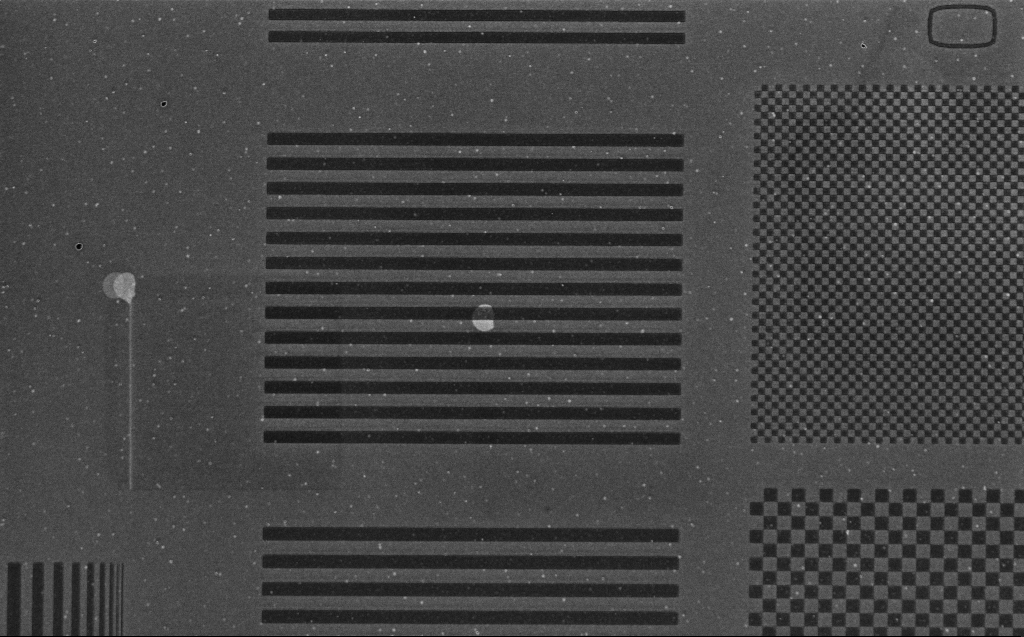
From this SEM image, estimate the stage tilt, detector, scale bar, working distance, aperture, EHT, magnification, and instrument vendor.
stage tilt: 0°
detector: InLens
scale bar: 10000 nm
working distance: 5 mm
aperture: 30 µm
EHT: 1.2 kV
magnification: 5.12 K X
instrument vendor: Zeiss